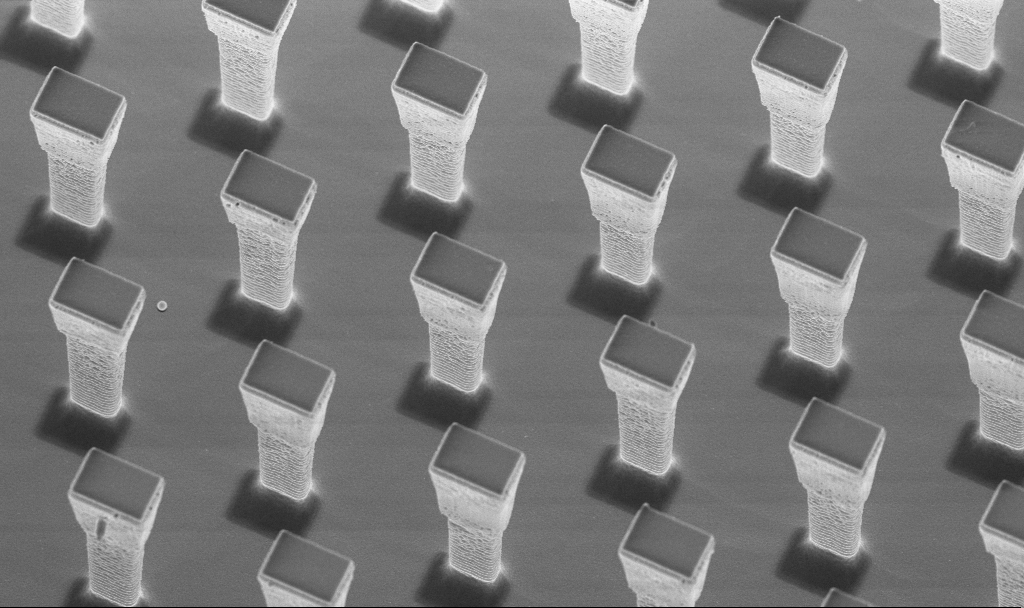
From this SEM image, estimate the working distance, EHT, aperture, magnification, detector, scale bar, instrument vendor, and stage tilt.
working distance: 4.3 mm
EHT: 5 kV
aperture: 30 µm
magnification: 6.42 K X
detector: InLens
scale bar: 10000 nm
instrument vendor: Zeiss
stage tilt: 20°